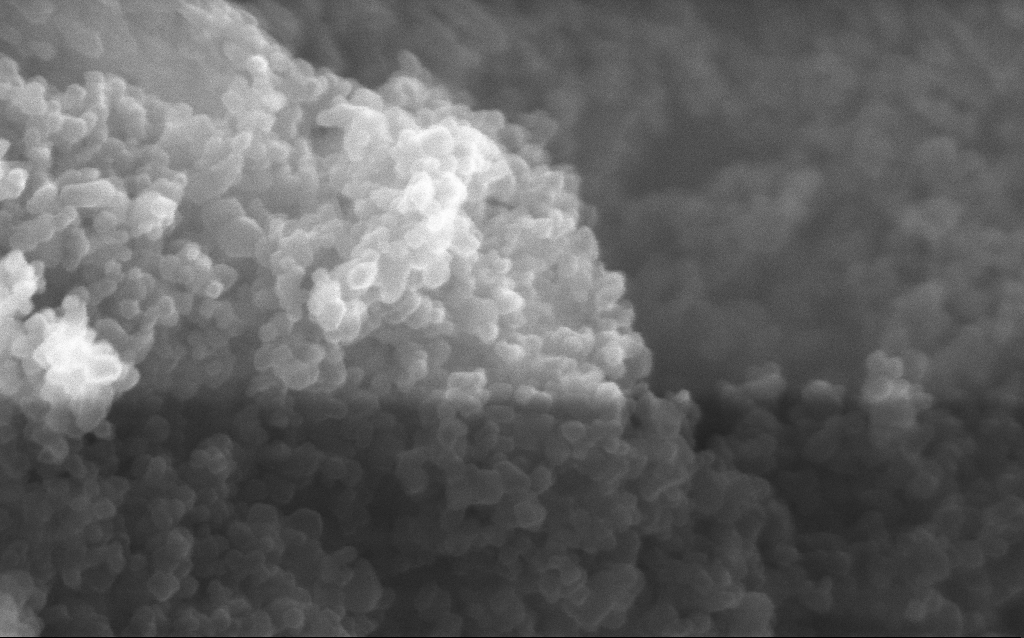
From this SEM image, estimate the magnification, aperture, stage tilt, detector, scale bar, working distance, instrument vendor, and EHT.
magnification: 348.1 K X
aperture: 30 µm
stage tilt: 0°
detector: InLens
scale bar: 100 nm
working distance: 2.7 mm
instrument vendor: Zeiss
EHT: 10 kV